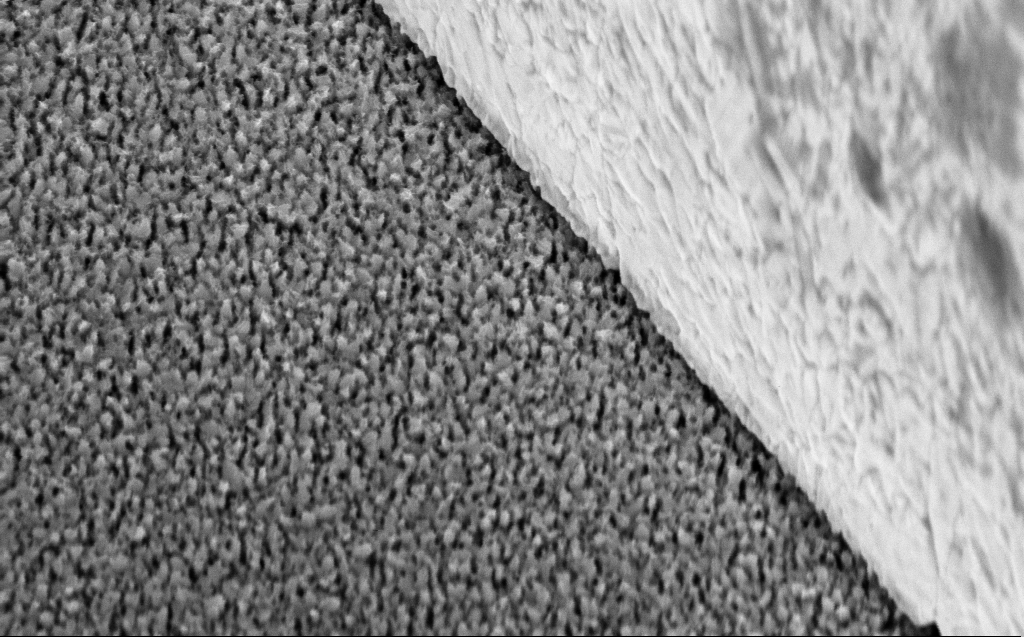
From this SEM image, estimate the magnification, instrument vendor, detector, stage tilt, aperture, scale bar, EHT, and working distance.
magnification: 62.78 K X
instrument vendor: Zeiss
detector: InLens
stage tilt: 45°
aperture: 30 µm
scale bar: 1000 nm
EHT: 5 kV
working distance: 6 mm